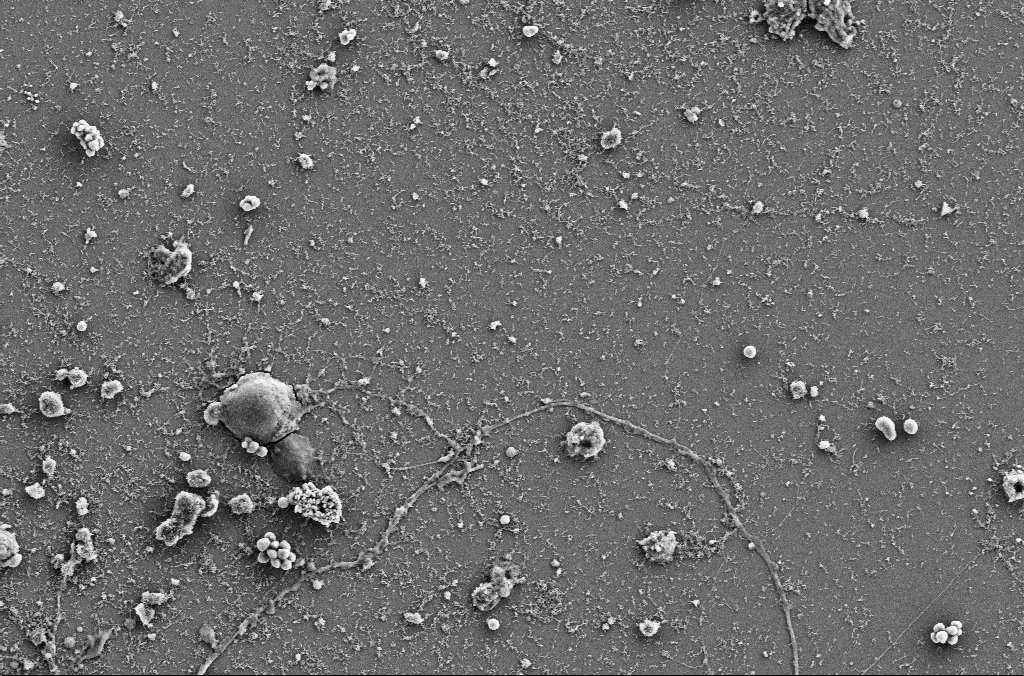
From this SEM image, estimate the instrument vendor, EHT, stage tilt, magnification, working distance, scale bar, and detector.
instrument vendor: Zeiss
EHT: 5 kV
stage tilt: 0°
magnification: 4 K X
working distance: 4 mm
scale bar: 10000 nm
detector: SE2